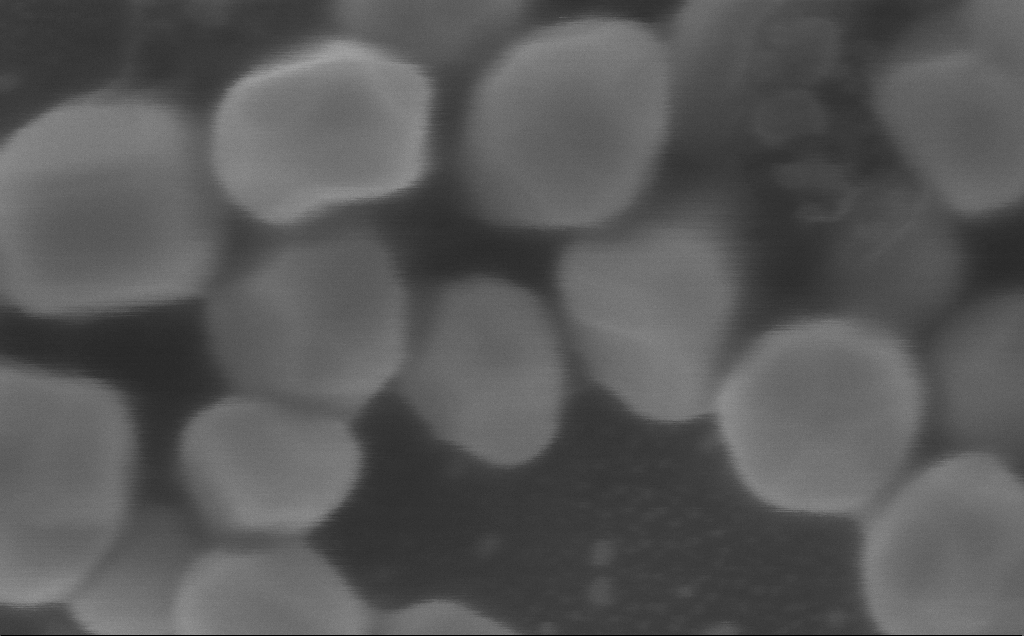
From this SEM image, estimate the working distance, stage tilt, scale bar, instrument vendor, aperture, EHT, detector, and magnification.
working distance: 3 mm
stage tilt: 0°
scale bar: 100 nm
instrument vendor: Zeiss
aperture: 30 µm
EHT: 5 kV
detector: InLens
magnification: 600 K X